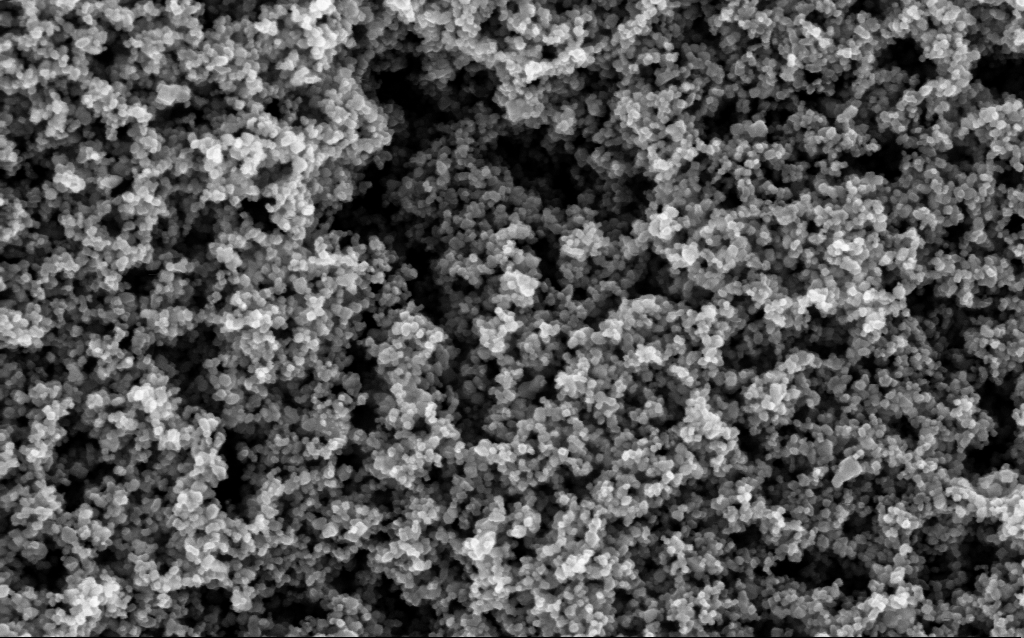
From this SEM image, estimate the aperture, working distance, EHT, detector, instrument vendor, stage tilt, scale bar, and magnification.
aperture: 30 µm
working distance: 5.3 mm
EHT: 5 kV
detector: InLens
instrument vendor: Zeiss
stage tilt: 0°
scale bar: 100 nm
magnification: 130 K X